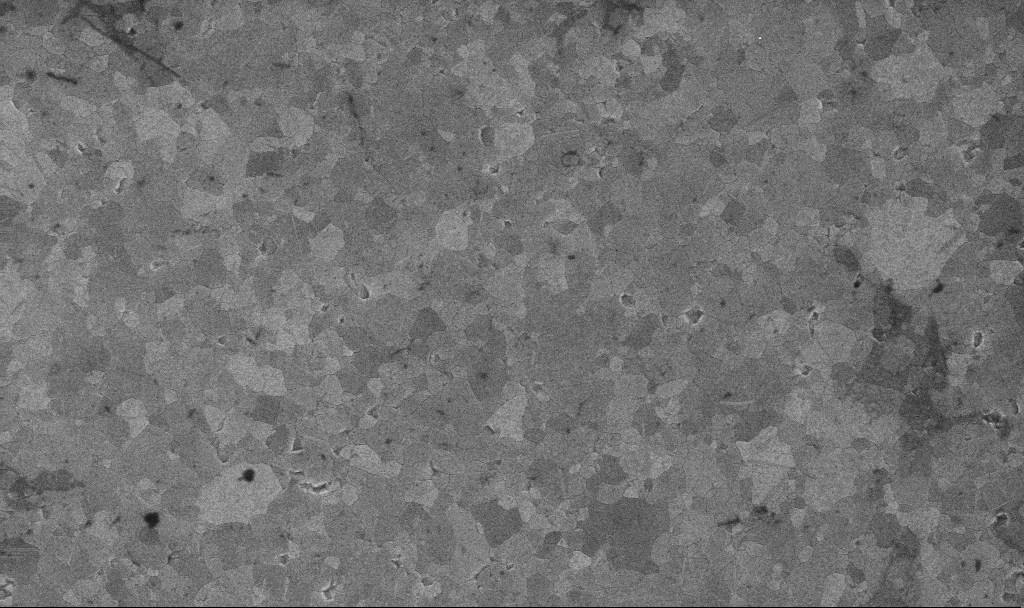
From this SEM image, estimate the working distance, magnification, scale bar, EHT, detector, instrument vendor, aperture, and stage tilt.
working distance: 3.1 mm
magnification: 46.56 K X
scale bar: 1000 nm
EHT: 10 kV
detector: InLens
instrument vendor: Zeiss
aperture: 30 µm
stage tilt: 0°